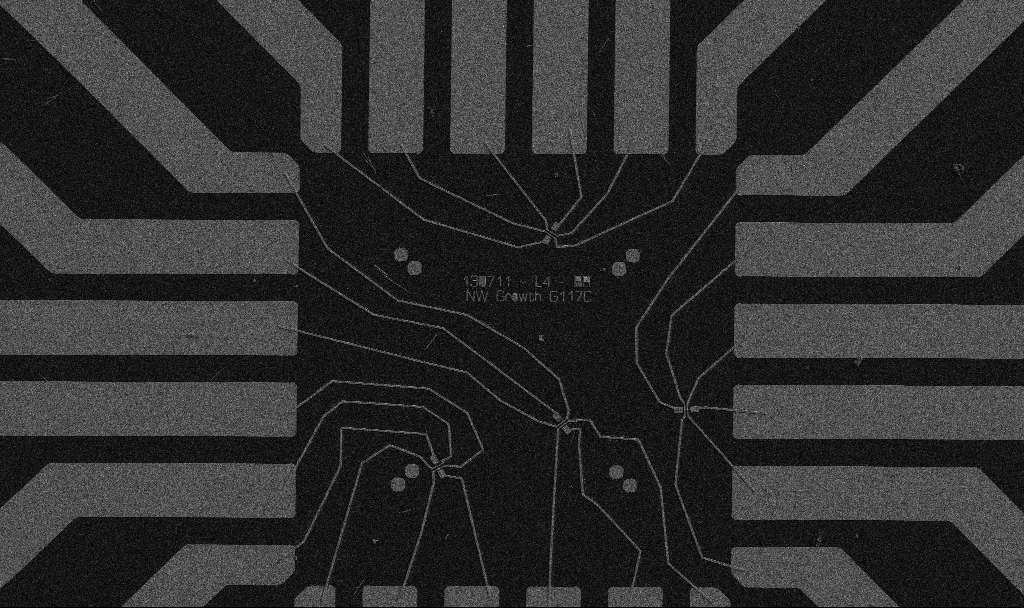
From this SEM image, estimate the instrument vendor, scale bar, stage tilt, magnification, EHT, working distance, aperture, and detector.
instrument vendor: Zeiss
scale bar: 20000 nm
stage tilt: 0°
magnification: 1 K X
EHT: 5 kV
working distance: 10.7 mm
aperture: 30 µm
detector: SE2